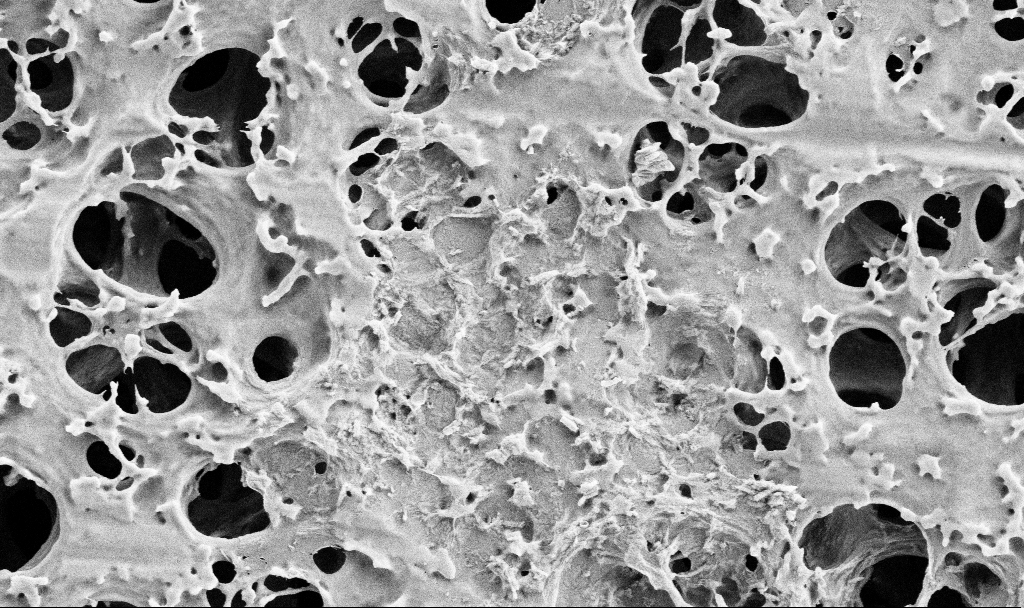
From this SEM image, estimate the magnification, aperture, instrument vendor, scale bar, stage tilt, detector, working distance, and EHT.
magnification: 25 K X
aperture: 30 µm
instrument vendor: Zeiss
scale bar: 2000 nm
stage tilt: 0°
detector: SE2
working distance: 3.6 mm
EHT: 2 kV